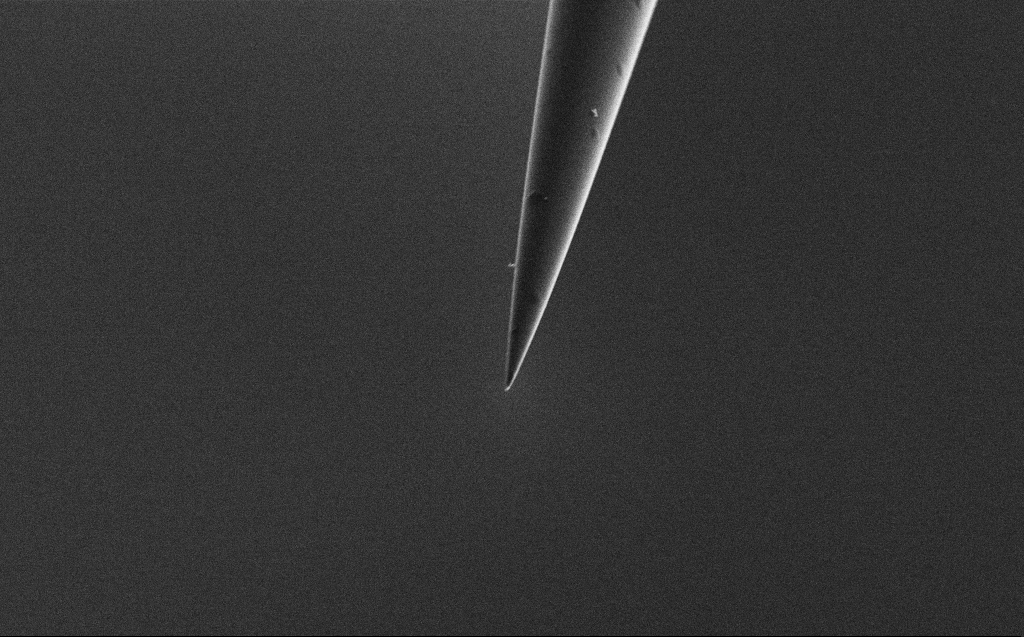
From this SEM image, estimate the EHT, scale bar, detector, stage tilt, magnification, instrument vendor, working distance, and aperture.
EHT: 2 kV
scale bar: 2000 nm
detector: SE2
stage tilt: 45°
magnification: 10 K X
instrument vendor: Zeiss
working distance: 4 mm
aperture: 30 µm